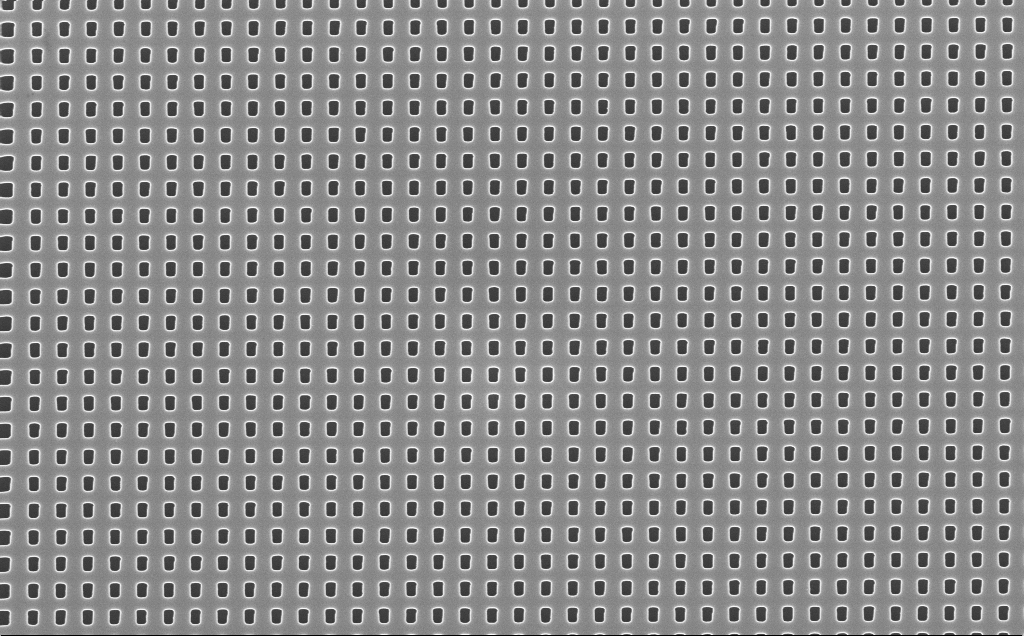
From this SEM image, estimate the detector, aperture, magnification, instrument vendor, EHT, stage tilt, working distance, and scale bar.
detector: InLens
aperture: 30 µm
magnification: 20 K X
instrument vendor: Zeiss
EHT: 10 kV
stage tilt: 0°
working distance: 5 mm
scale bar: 1000 nm